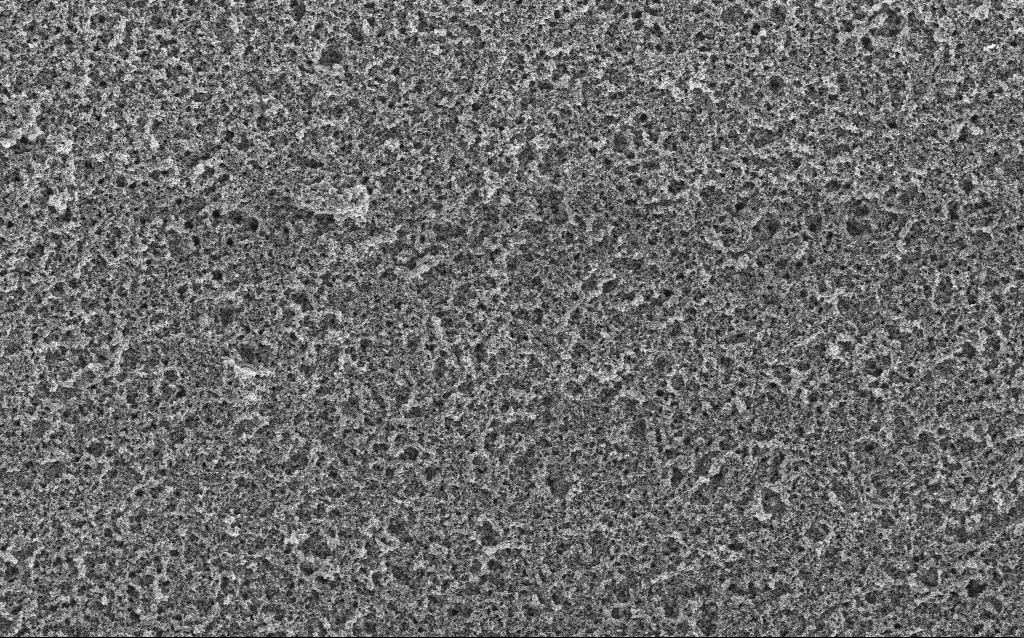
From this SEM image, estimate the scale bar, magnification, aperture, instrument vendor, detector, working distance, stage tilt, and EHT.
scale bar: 10000 nm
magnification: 6.61 K X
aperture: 30 µm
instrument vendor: Zeiss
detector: InLens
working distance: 4.4 mm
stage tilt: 0°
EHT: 5 kV